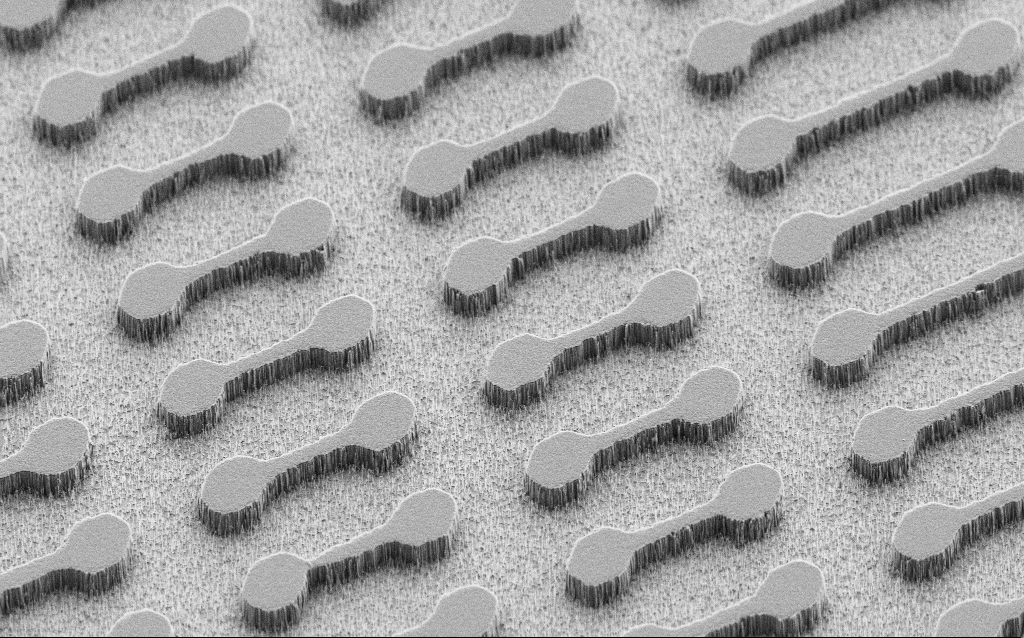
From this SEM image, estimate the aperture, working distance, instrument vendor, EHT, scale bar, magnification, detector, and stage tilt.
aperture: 30 µm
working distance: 7 mm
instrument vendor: Zeiss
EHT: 3 kV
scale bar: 10000 nm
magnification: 1.5 K X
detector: SE2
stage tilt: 45°